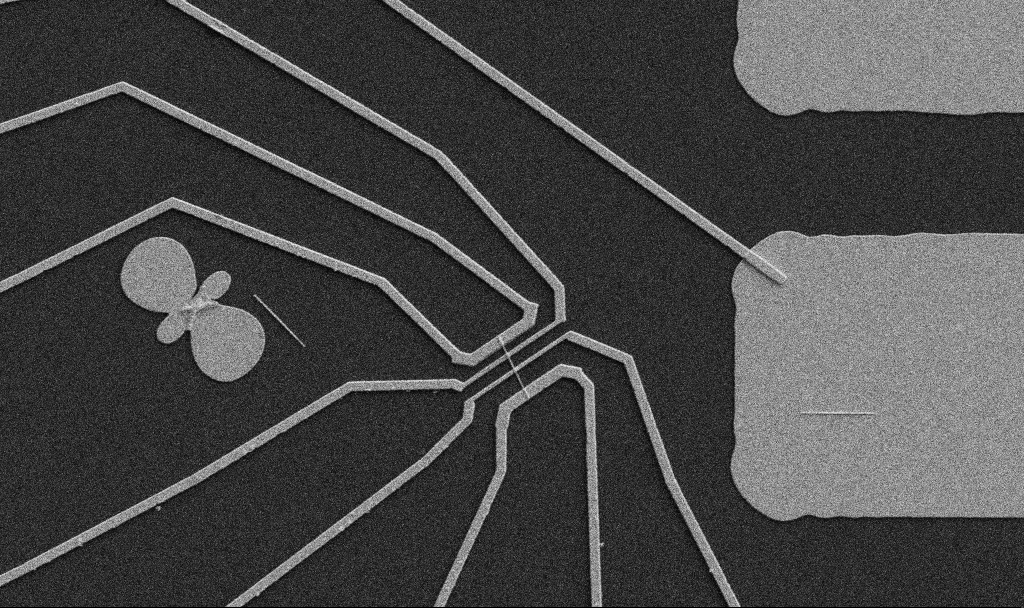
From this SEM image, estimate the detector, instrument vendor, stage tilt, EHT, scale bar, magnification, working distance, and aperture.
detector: SE2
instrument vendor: Zeiss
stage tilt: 0°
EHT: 5 kV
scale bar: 10000 nm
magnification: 5 K X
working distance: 10.7 mm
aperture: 30 µm